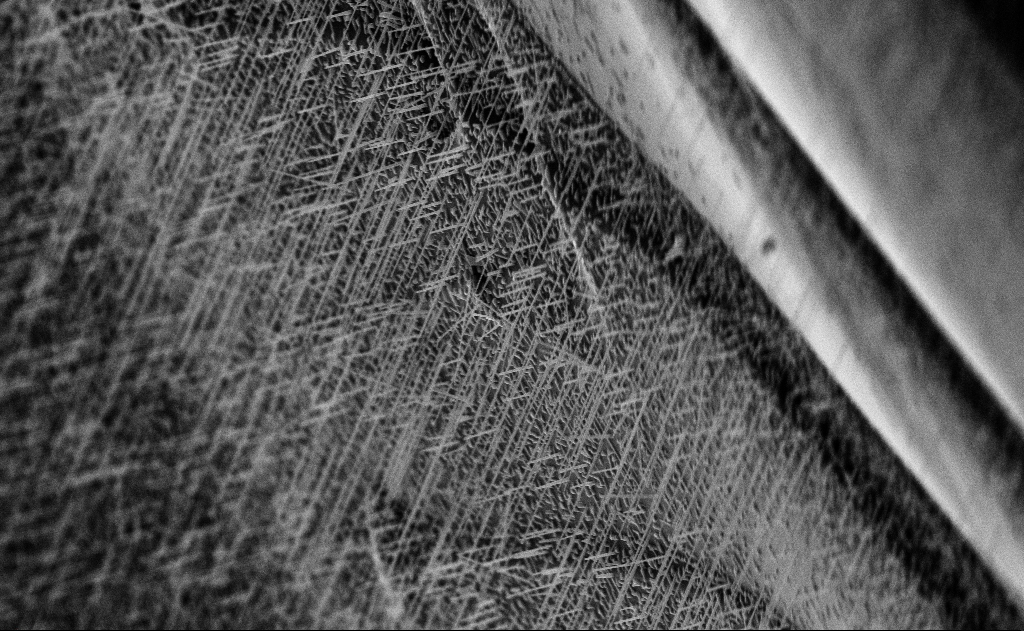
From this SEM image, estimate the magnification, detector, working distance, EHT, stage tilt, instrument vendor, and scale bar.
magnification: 10 K X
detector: InLens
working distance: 14 mm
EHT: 10 kV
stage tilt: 0°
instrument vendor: Zeiss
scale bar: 2000 nm